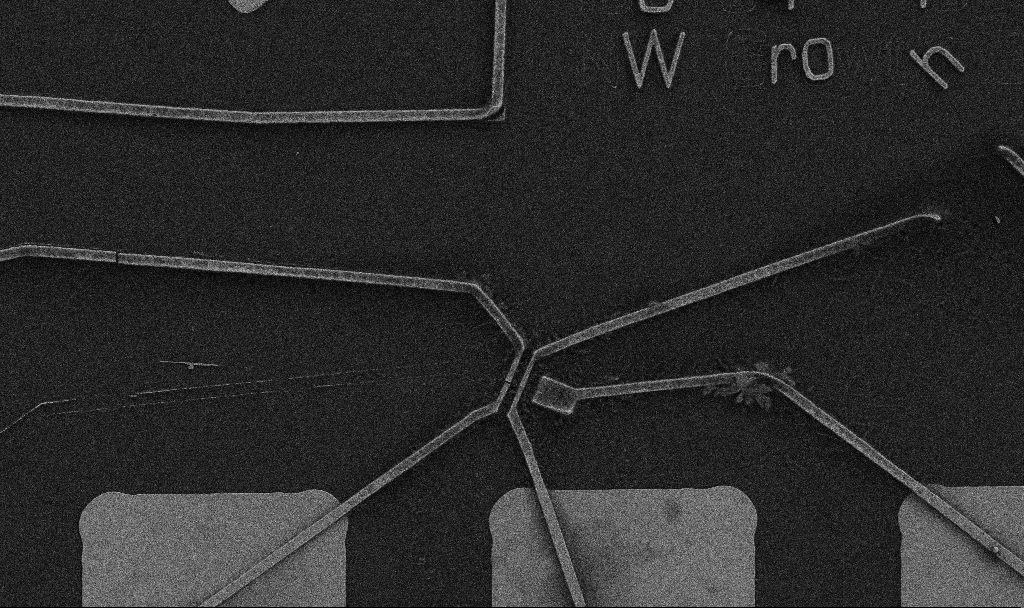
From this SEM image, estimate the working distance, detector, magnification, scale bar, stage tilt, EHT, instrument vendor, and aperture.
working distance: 9.7 mm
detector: SE2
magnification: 5 K X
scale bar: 10000 nm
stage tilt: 0°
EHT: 5 kV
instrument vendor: Zeiss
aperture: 30 µm